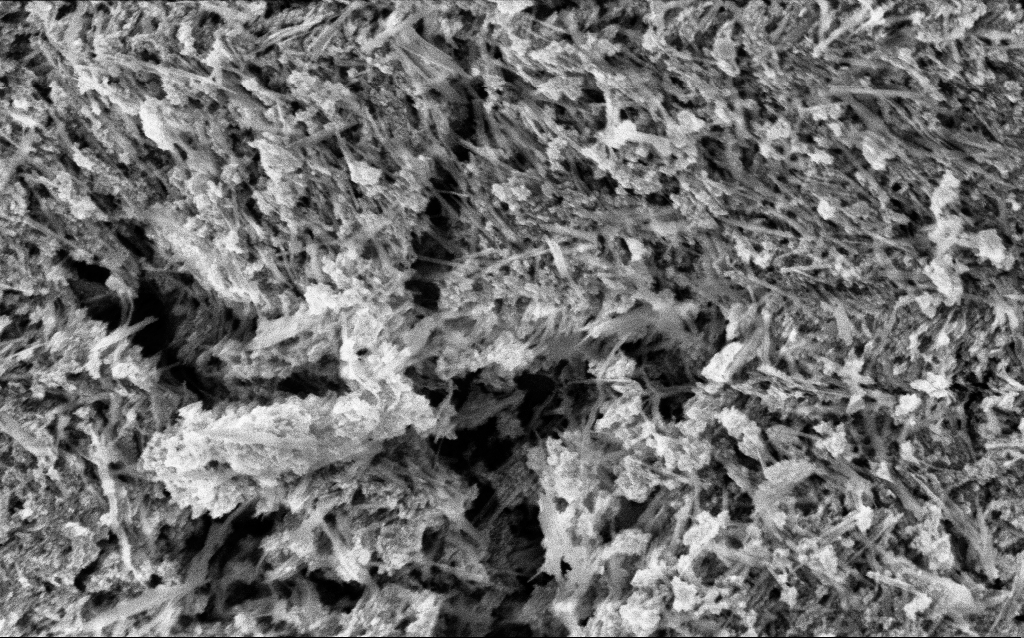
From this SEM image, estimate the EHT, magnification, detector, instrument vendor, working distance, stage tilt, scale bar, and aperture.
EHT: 3 kV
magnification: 101.19 K X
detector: InLens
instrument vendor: Zeiss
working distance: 5.1 mm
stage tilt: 0°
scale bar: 200 nm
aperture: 30 µm